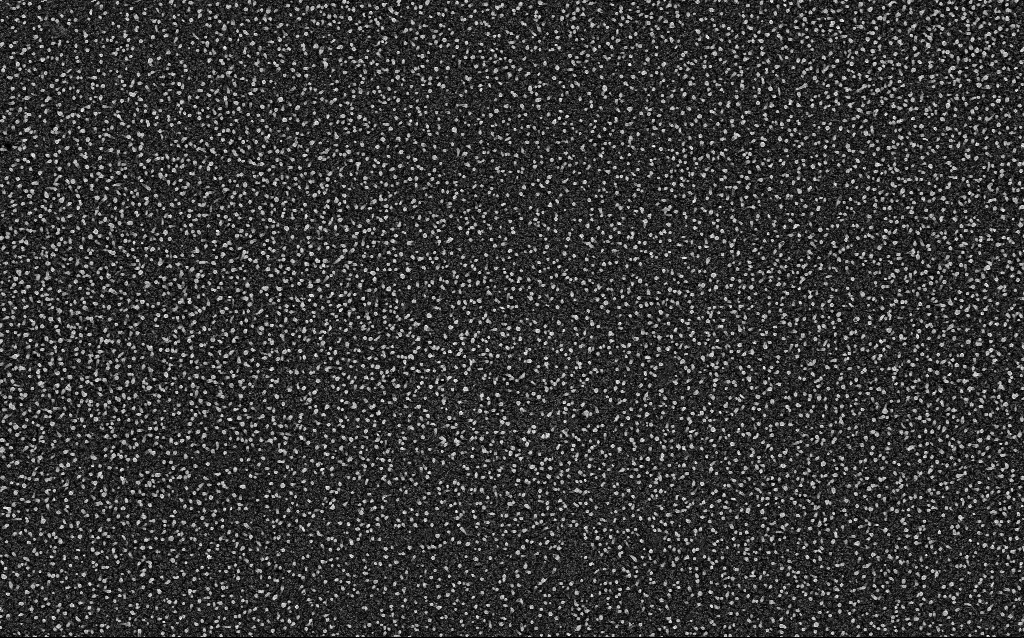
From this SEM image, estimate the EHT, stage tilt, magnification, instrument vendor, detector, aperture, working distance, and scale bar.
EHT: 5 kV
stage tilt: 0°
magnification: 20 K X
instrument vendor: Zeiss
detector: InLens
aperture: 30 µm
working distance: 1.9 mm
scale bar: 1000 nm